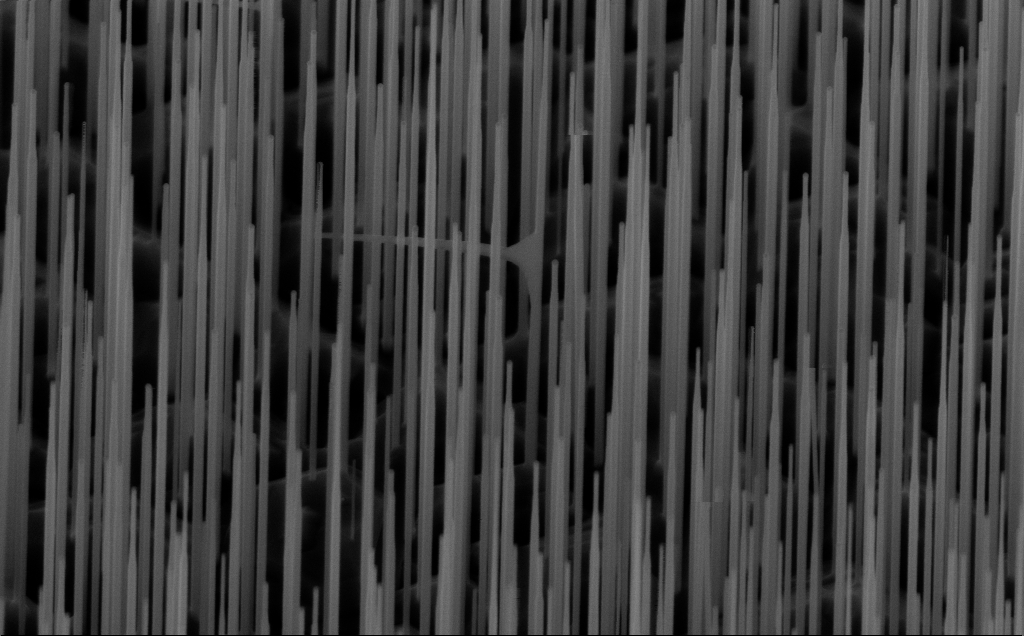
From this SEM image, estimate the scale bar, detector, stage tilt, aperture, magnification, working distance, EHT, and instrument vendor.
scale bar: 1000 nm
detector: InLens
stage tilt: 45°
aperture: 30 µm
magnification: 40 K X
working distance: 6 mm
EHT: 10 kV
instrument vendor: Zeiss